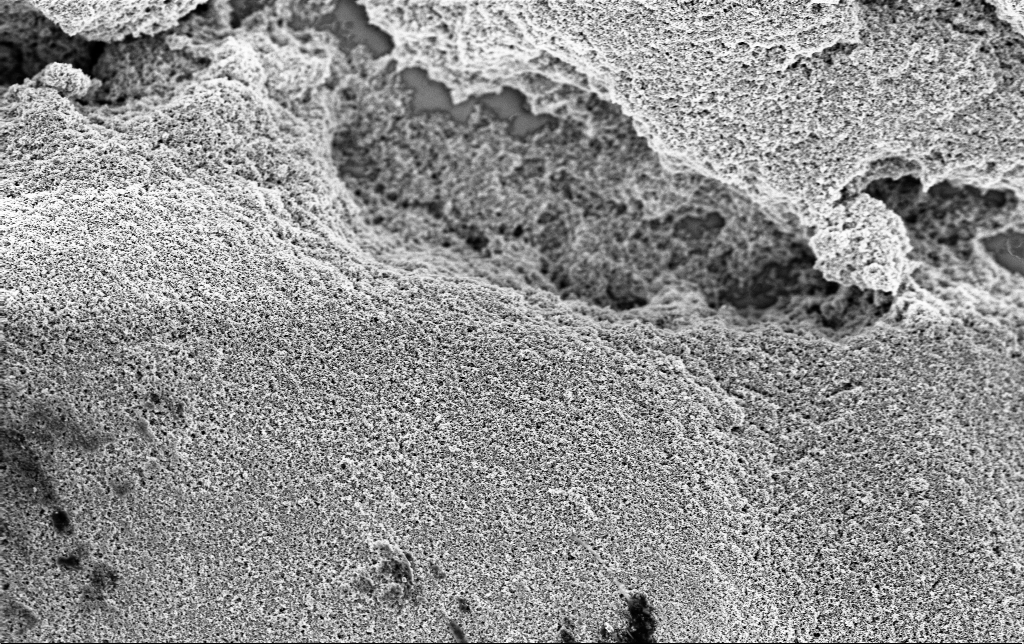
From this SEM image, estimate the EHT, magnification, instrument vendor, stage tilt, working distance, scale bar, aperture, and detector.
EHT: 3 kV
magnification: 10 K X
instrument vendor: Zeiss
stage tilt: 0°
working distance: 2.8 mm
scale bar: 2000 nm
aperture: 30 µm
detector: InLens